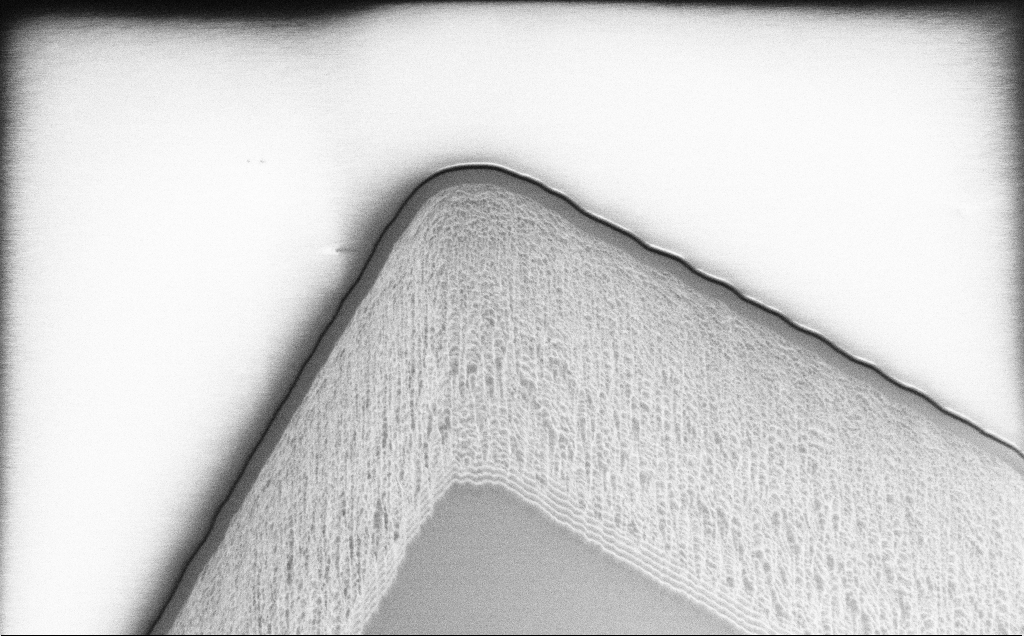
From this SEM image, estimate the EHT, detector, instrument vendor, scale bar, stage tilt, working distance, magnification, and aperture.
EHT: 1.2 kV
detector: InLens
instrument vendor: Zeiss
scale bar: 2000 nm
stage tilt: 45°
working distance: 7 mm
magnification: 7.8 K X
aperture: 30 µm